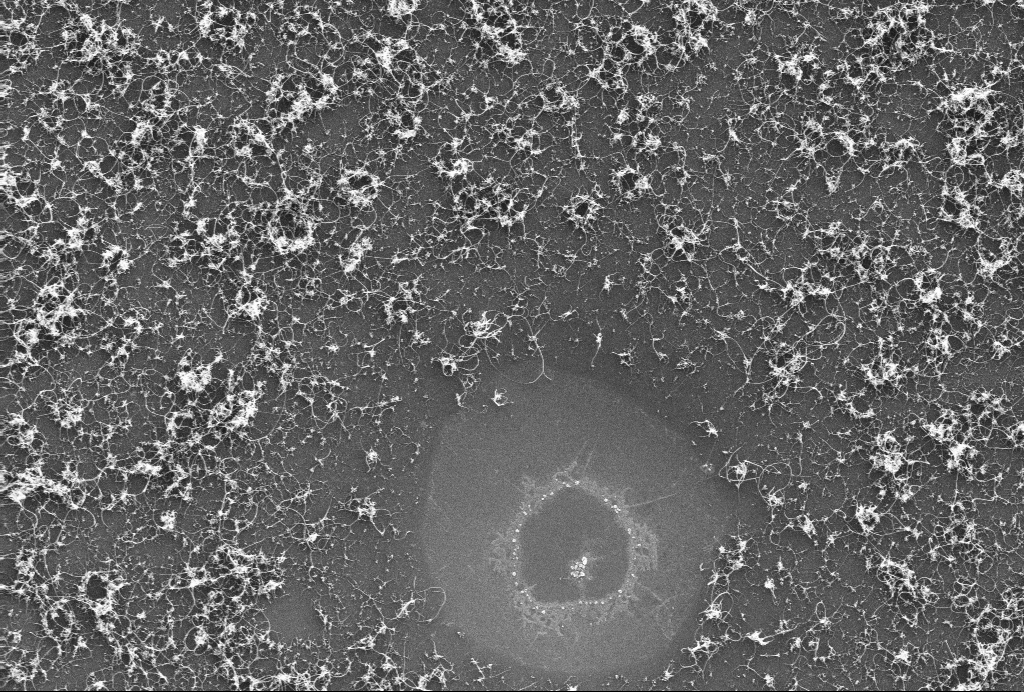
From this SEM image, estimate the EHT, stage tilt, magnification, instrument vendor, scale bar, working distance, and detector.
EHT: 10 kV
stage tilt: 0°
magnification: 33.17 K X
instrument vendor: Zeiss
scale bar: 1000 nm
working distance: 3.1 mm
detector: InLens